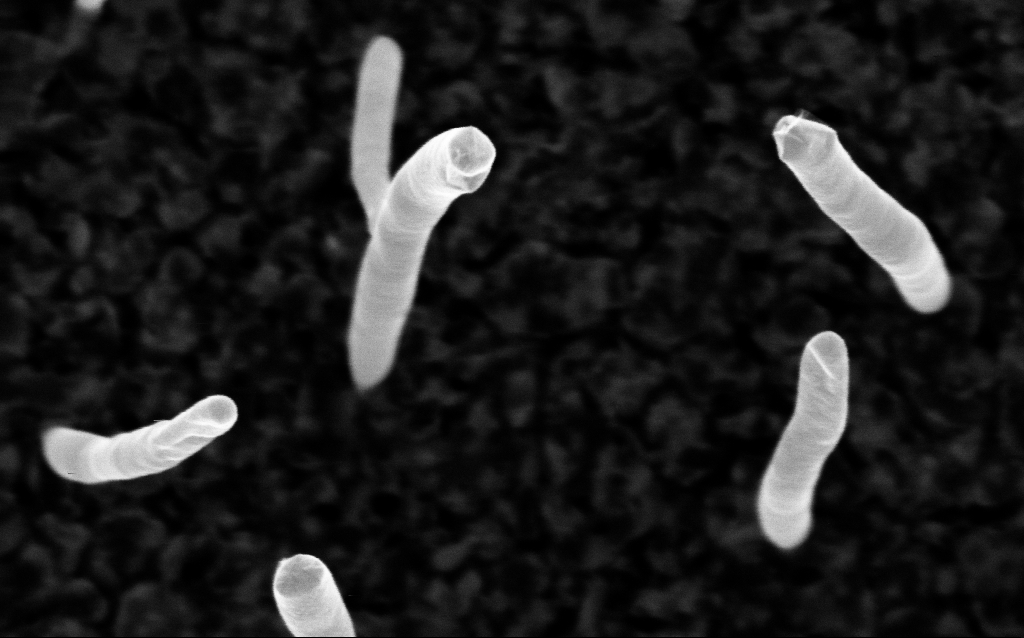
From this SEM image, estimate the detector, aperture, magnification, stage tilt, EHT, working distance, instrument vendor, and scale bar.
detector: InLens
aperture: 30 µm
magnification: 200 K X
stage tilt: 0°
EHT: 5 kV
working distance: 1.8 mm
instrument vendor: Zeiss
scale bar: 100 nm